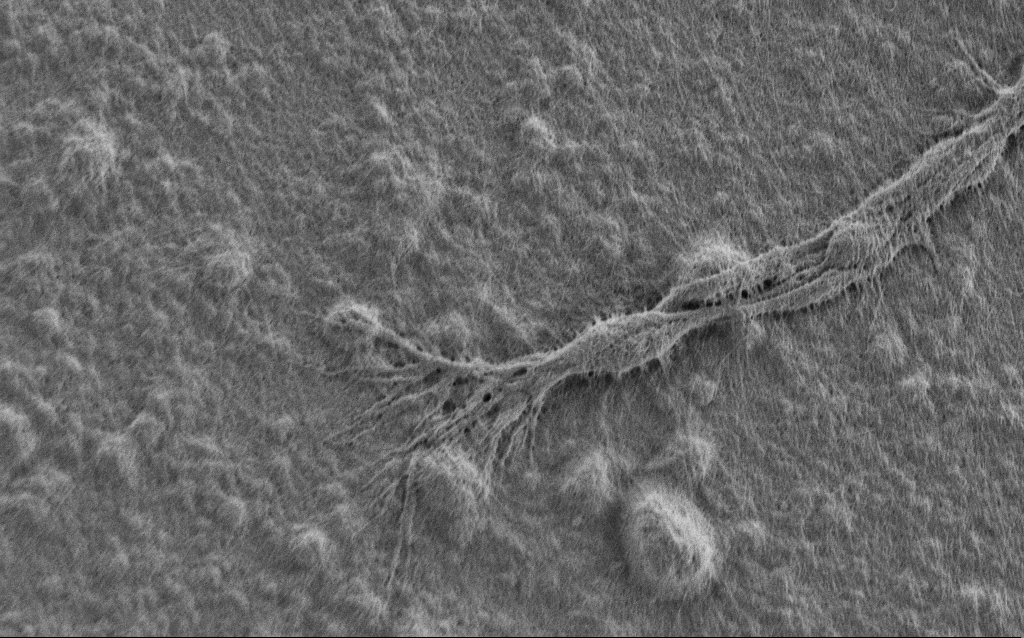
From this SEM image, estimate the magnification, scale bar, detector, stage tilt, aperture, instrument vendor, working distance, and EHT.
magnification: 10 K X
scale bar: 2000 nm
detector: SE2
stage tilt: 0°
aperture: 30 µm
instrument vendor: Zeiss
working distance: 7 mm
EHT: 0.9 kV